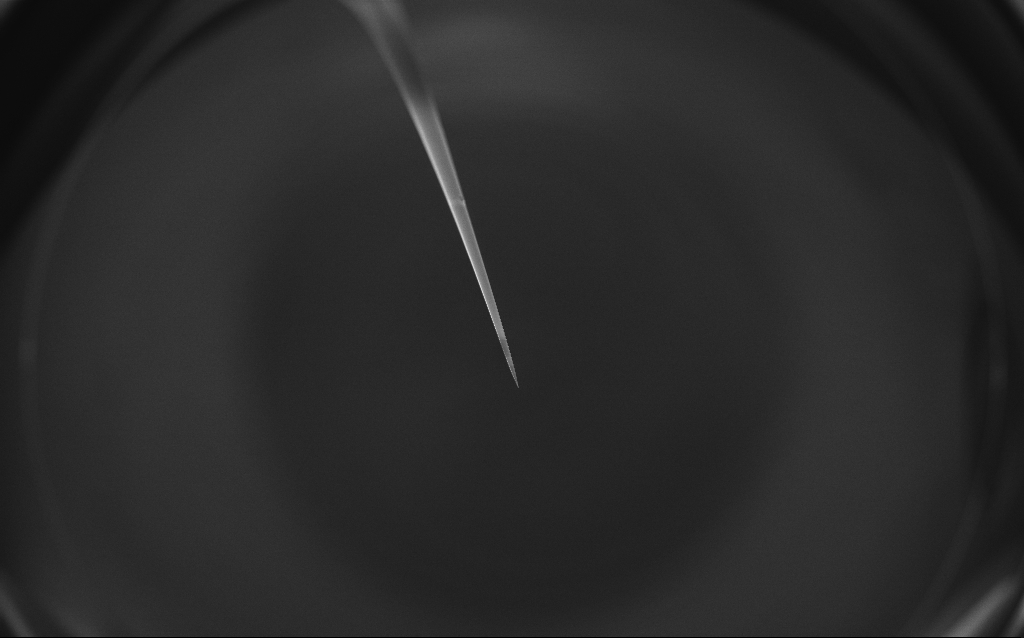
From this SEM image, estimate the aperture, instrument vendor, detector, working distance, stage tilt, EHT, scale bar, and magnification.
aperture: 30 µm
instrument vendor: Zeiss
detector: InLens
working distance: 7 mm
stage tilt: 45°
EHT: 1 kV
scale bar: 200000 nm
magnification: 0.1 K X